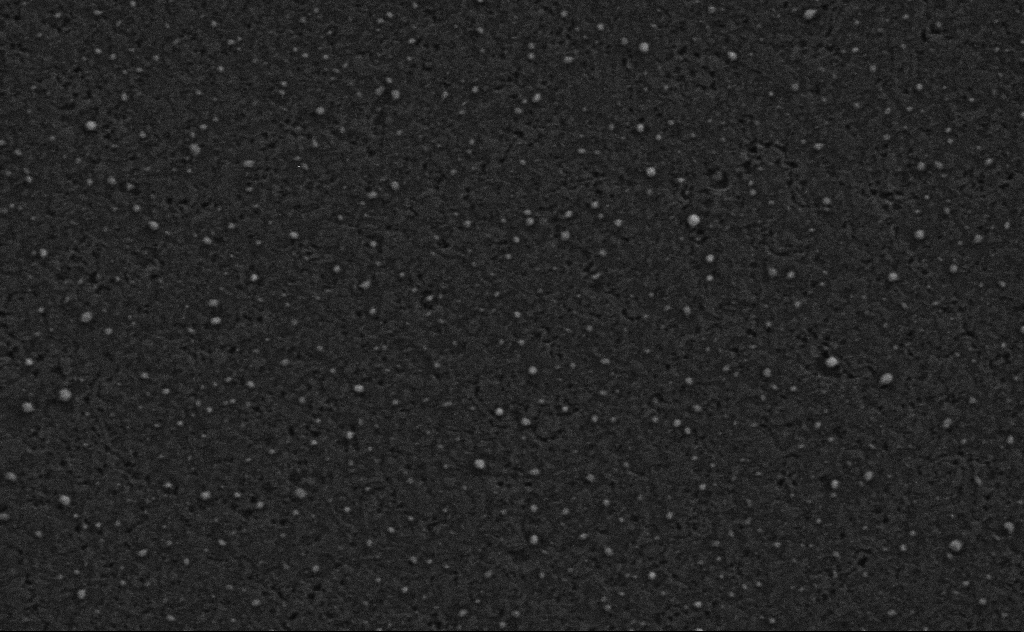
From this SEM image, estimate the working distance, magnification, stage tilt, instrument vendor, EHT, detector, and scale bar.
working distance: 4 mm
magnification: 80 K X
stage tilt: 0°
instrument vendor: Zeiss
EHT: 3 kV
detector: SE2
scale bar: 200 nm